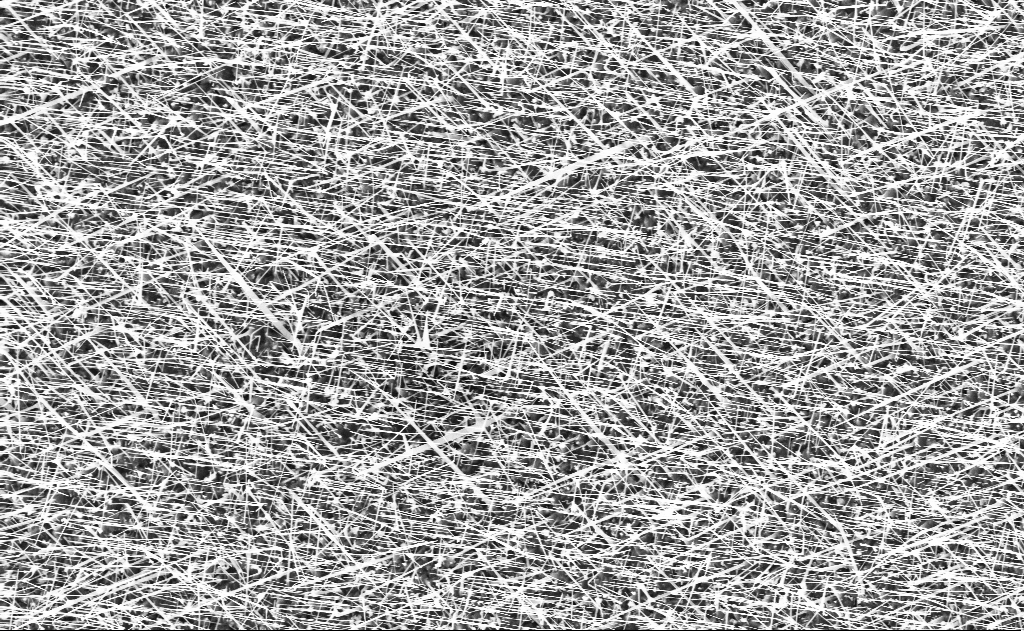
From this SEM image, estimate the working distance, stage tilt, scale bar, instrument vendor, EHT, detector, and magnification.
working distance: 10 mm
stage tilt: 0°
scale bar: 2000 nm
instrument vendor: Zeiss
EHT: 10 kV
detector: InLens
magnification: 10 K X